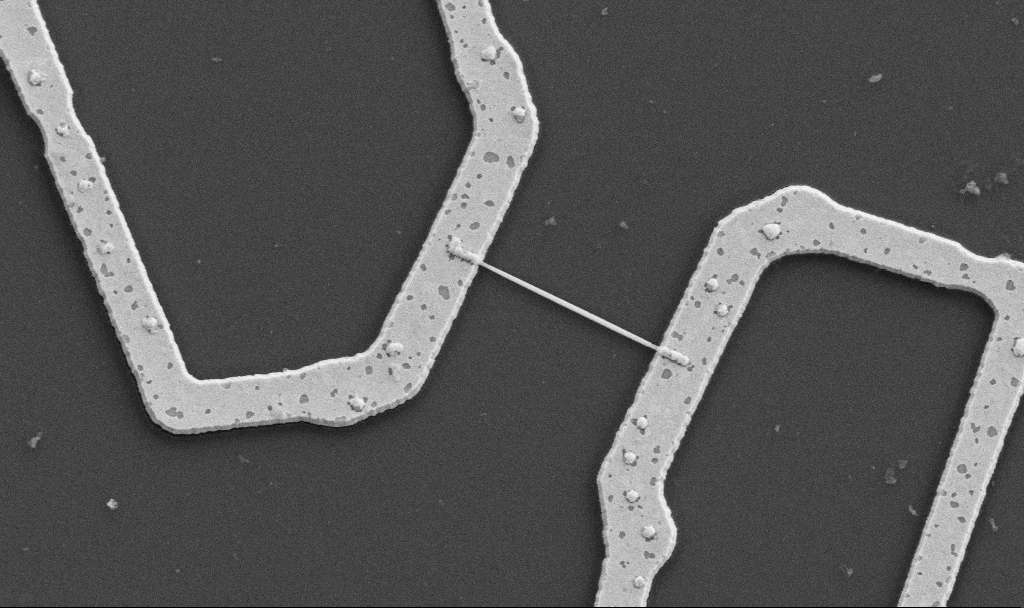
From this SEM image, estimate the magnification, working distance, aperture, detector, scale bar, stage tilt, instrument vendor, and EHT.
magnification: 20 K X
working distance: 10.7 mm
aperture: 30 µm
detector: SE2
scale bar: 1000 nm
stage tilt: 0°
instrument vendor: Zeiss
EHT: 5 kV